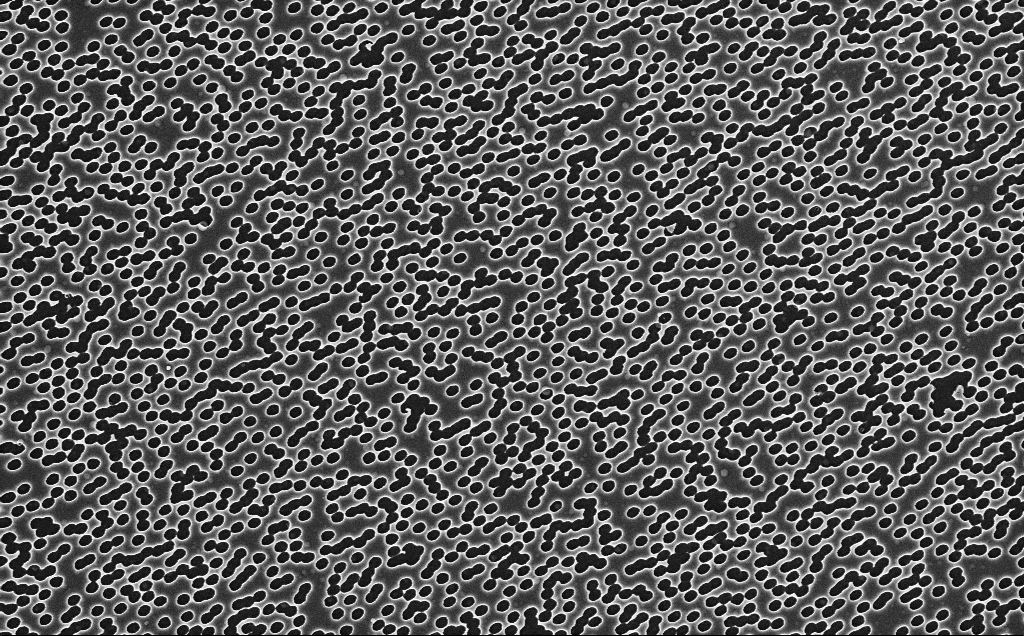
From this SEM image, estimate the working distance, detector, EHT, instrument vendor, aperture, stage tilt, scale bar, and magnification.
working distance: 2.5 mm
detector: InLens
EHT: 3 kV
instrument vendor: Zeiss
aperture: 30 µm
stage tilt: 0°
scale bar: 2000 nm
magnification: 17.59 K X